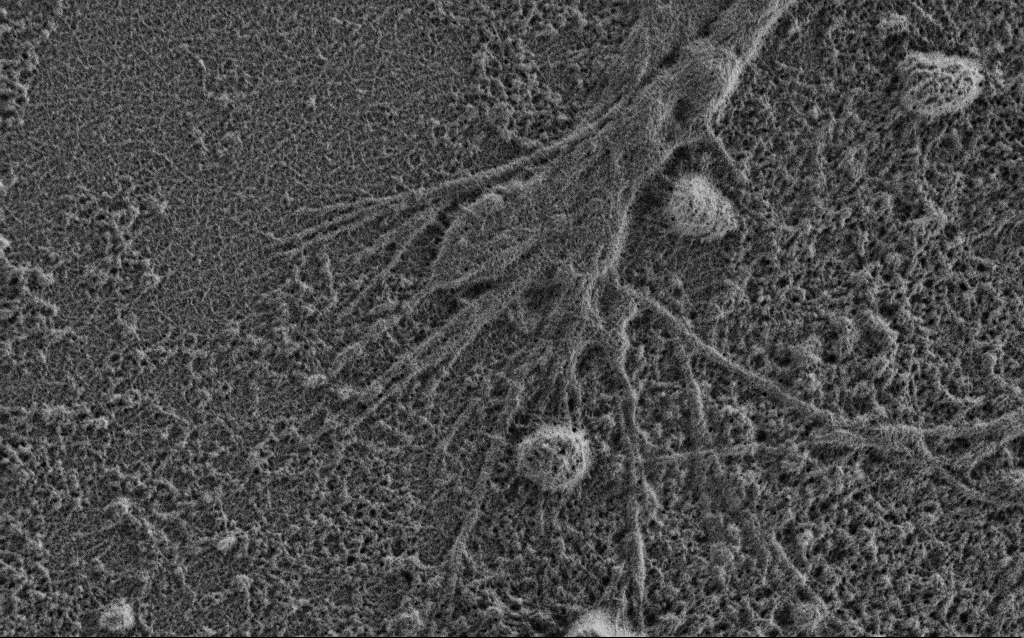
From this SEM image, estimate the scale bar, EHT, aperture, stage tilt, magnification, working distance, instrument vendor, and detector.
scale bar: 2000 nm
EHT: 0.9 kV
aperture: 30 µm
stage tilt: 0°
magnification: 15 K X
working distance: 3.4 mm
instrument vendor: Zeiss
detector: SE2